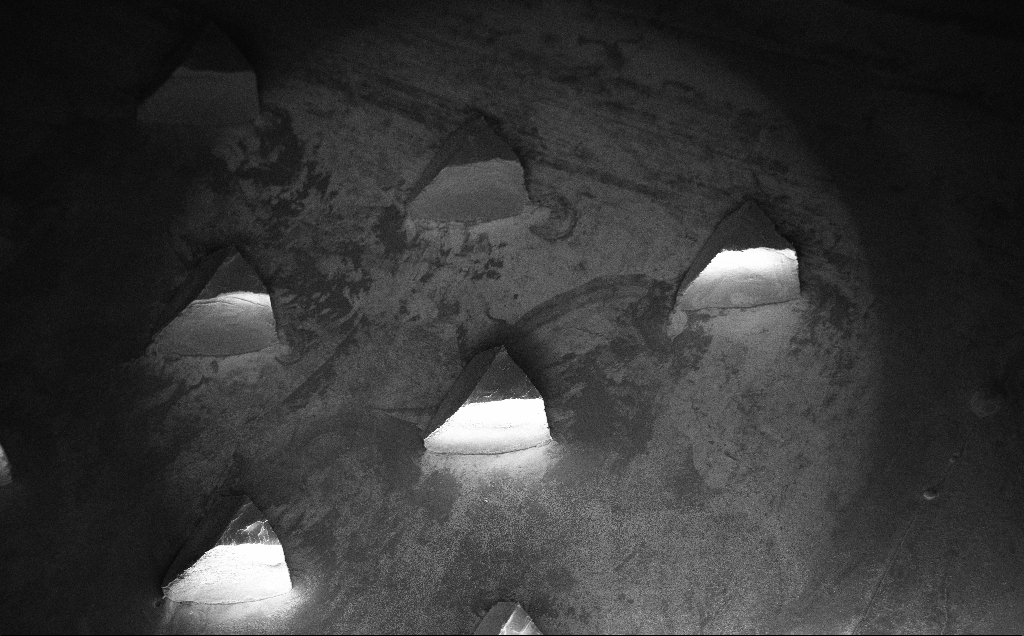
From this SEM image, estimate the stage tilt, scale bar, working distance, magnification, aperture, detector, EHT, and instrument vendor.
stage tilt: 30°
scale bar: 200000 nm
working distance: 9 mm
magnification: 0.08 K X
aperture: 30 µm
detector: InLens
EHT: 10 kV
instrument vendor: Zeiss